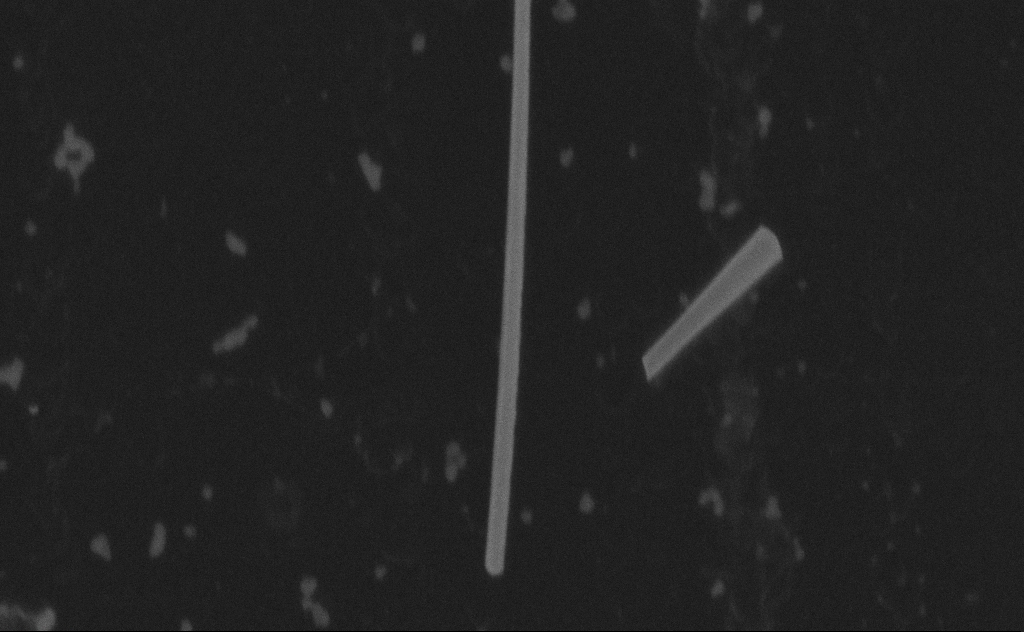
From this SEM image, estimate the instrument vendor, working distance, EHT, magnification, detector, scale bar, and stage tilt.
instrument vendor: Zeiss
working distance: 9 mm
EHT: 20 kV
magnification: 107.68 K X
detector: SE2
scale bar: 200 nm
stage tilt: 0°